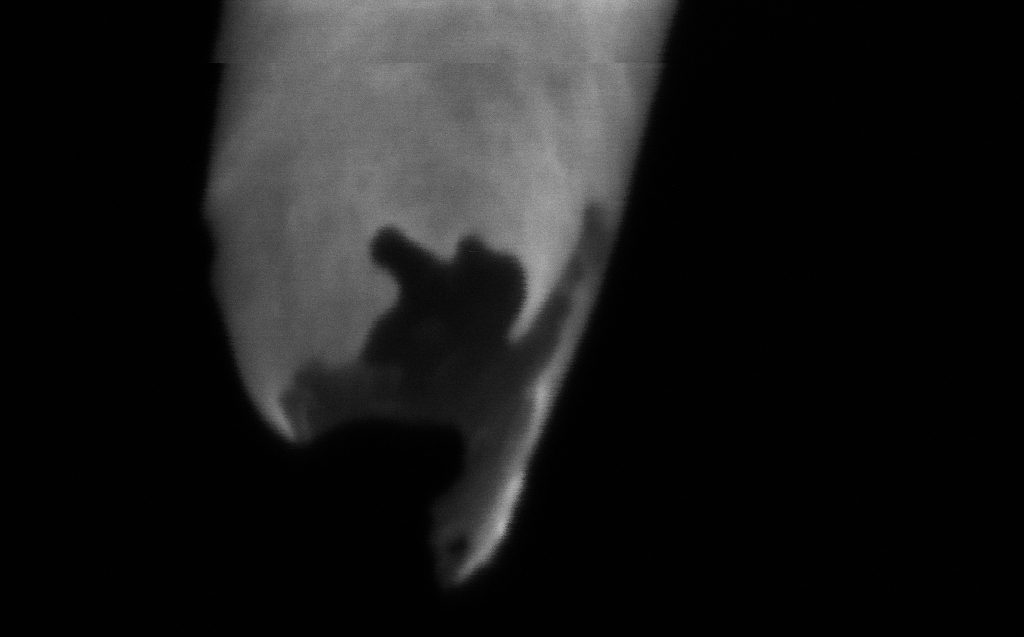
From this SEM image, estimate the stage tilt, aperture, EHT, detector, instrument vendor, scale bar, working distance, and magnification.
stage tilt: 45°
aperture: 30 µm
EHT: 2 kV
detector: InLens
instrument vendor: Zeiss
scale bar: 20 nm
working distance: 4 mm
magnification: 763.13 K X